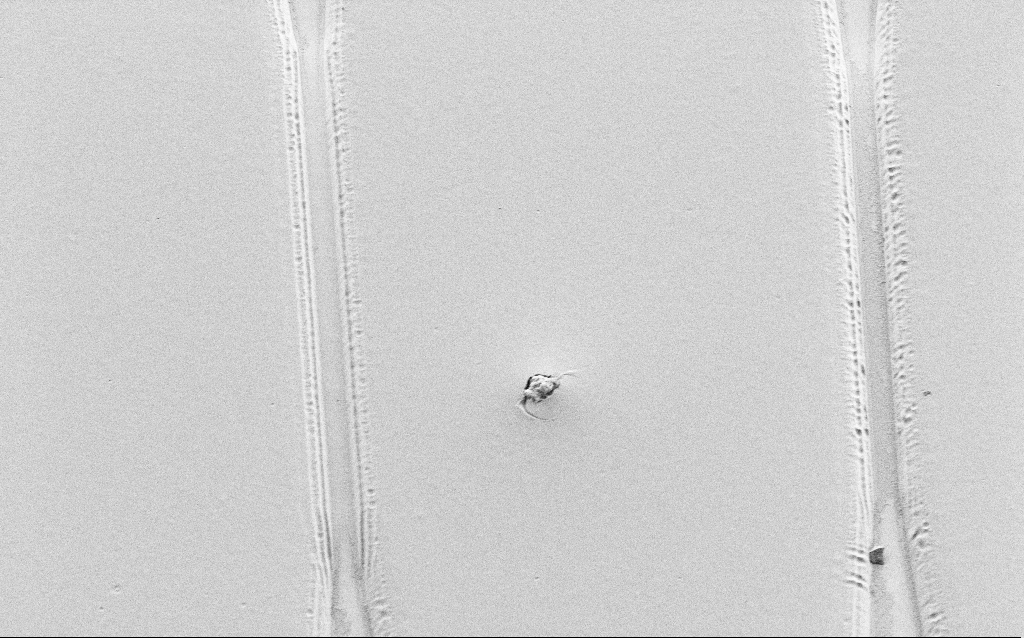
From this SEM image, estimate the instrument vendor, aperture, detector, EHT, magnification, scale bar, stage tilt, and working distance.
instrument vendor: Zeiss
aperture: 30 µm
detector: SE2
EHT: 1 kV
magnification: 1.93 K X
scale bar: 20000 nm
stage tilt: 36°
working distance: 6 mm